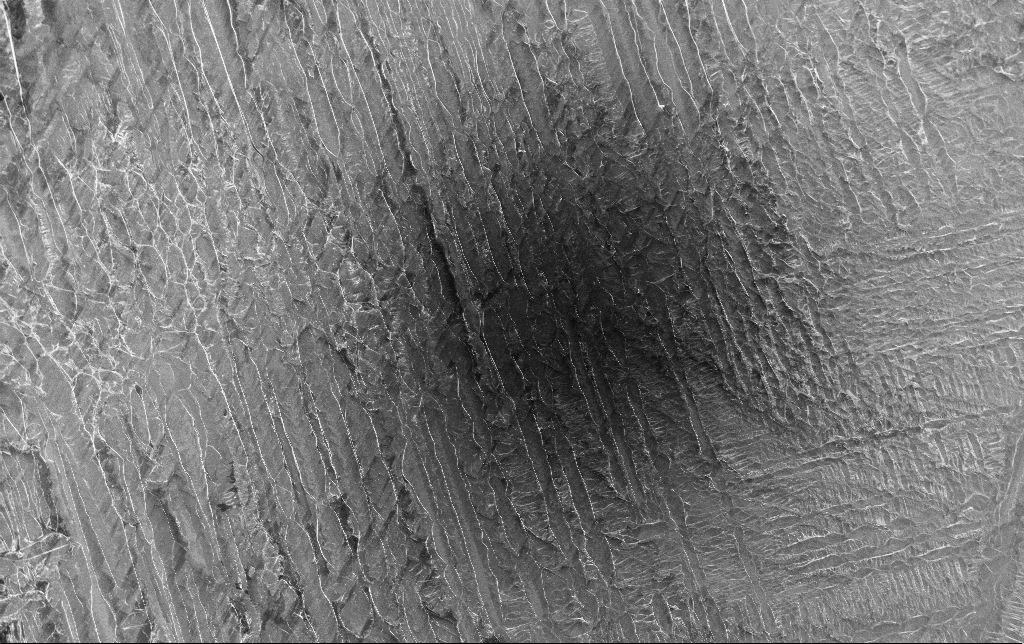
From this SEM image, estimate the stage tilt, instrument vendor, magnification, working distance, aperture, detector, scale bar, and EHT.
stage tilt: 0°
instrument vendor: Zeiss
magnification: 0.252 K X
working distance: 3 mm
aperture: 30 µm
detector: InLens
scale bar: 100000 nm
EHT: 5 kV